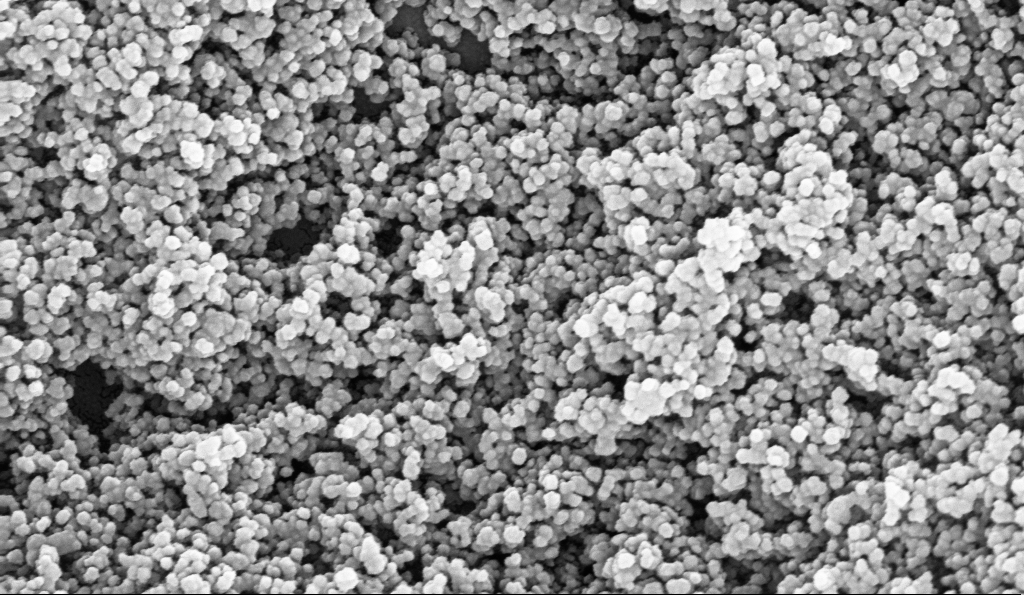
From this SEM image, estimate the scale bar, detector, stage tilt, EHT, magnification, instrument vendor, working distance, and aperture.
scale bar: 200 nm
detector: InLens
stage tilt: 0°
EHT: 10 kV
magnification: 135 K X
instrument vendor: Zeiss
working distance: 5.1 mm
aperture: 30 µm